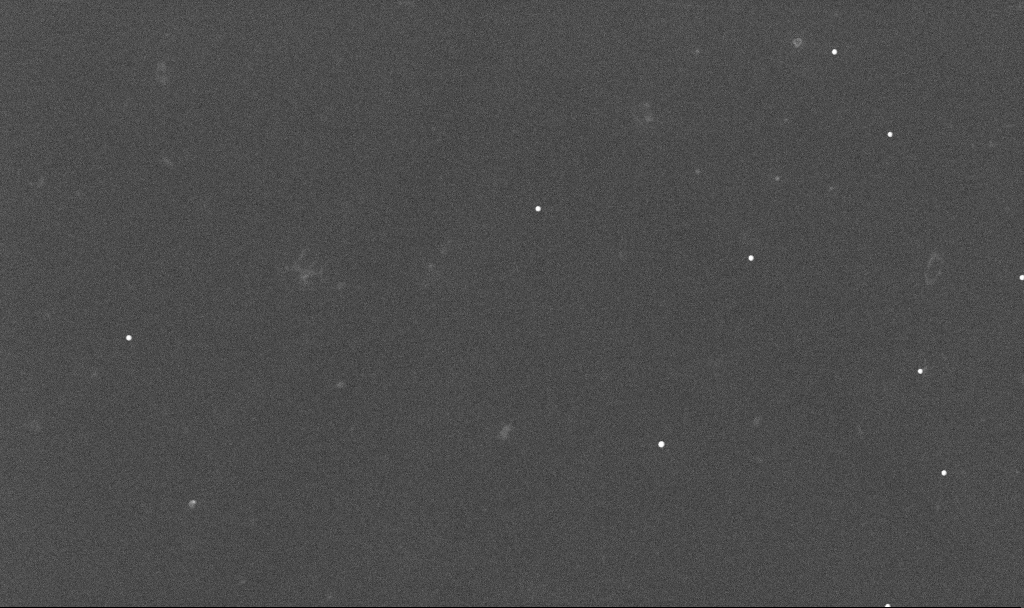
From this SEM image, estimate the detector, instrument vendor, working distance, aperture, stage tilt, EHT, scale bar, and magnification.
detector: InLens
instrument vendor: Zeiss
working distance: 3.4 mm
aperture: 30 µm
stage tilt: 0°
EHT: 10 kV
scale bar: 1000 nm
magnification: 20 K X